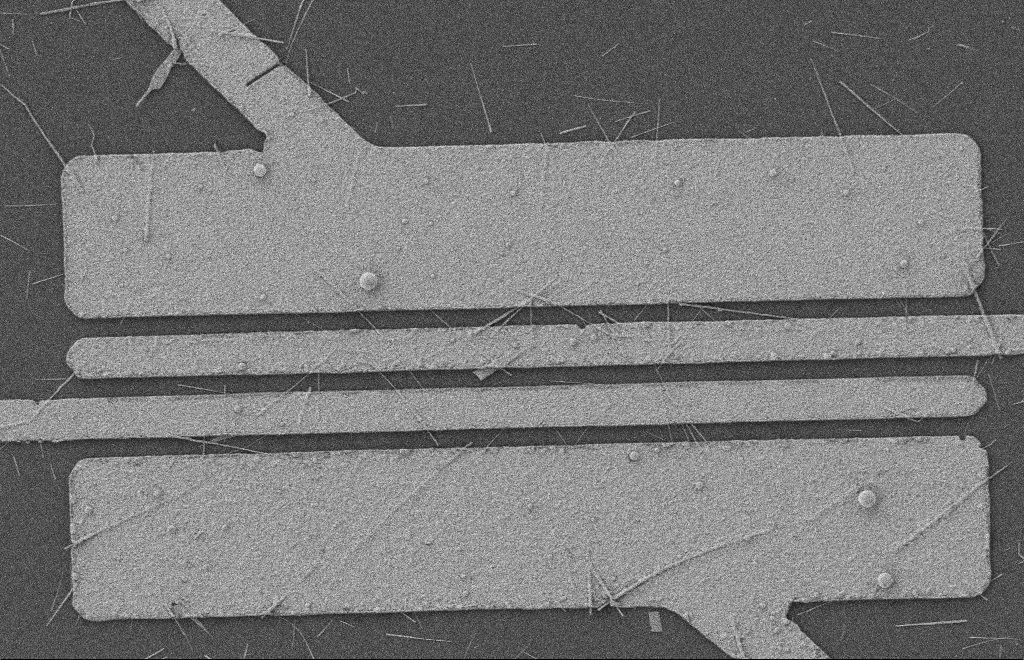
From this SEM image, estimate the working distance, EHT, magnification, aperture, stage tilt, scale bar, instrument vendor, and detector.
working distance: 8 mm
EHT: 2 kV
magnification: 5.54 K X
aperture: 20 µm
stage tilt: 0°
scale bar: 2000 nm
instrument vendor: Zeiss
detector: SE2